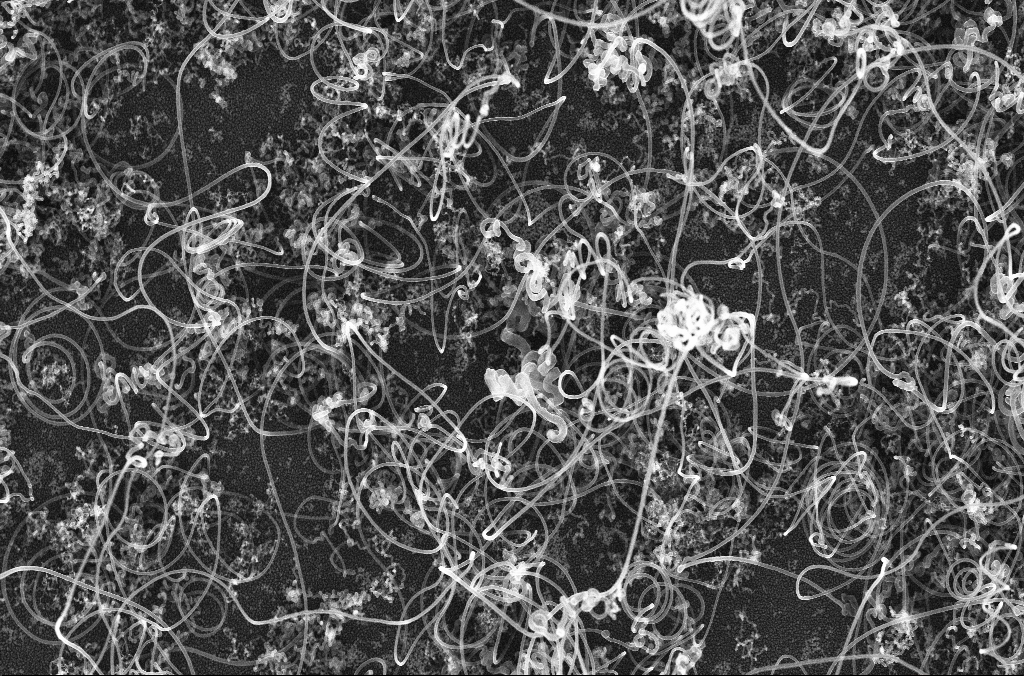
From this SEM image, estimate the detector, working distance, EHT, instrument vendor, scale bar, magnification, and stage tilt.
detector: InLens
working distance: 4.2 mm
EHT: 20 kV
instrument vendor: Zeiss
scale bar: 2000 nm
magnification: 10 K X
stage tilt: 0°